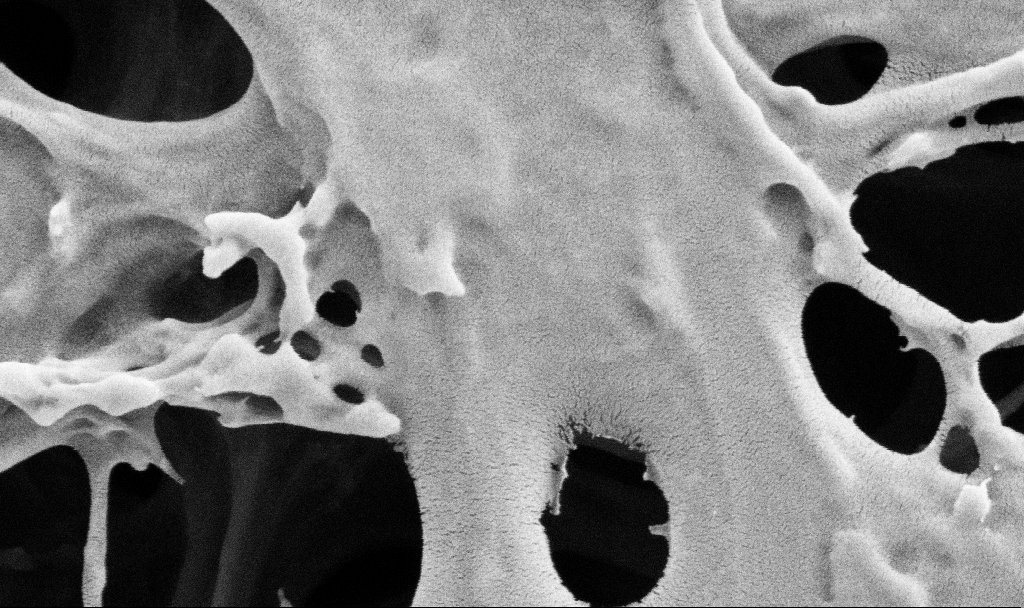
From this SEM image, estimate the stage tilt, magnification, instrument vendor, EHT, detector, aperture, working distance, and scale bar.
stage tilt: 0°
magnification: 100 K X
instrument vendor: Zeiss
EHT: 2 kV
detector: SE2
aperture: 30 µm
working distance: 3.7 mm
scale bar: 200 nm